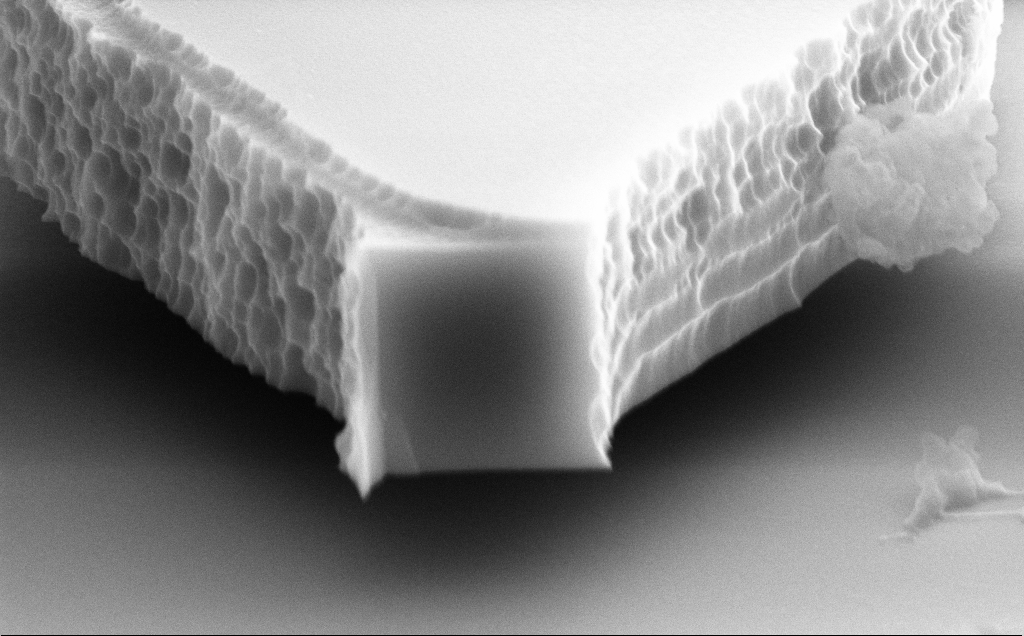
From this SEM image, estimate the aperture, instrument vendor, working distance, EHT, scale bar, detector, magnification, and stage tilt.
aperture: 30 µm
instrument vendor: Zeiss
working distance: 9 mm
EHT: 10 kV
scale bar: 1000 nm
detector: SE2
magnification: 39.42 K X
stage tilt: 70°